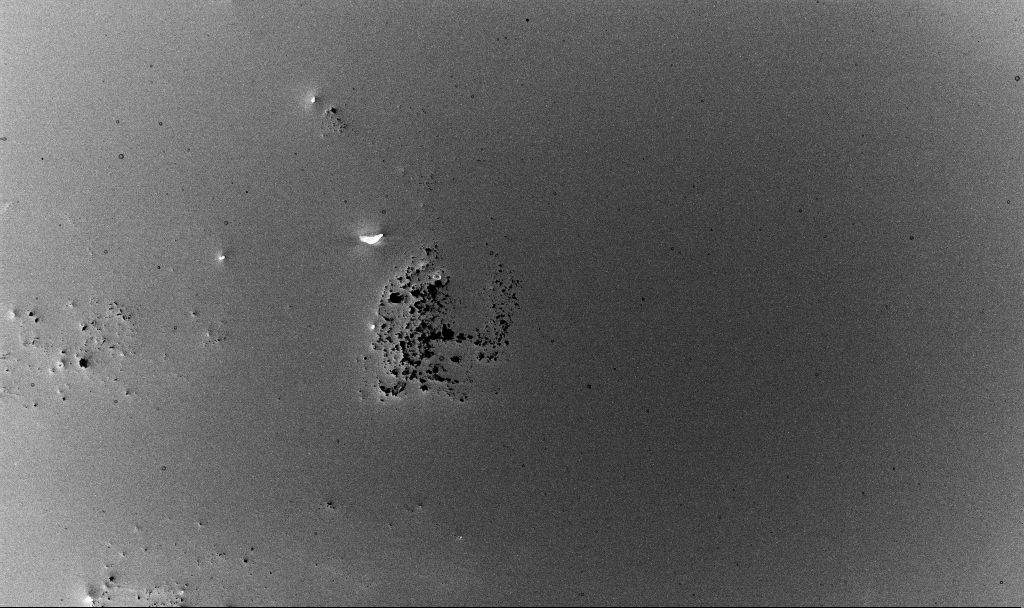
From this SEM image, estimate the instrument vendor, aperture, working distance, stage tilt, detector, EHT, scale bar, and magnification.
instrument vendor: Zeiss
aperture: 30 µm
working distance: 3.3 mm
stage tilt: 0°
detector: InLens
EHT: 10 kV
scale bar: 20000 nm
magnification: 1 K X